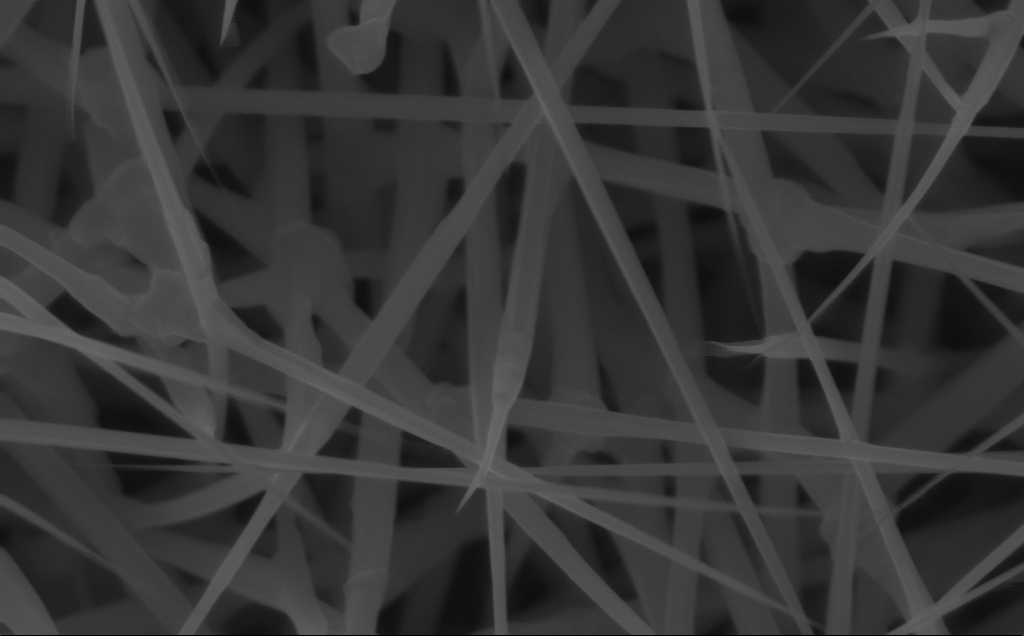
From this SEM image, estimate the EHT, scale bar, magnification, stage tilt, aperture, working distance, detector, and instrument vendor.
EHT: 10 kV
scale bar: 200 nm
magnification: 100 K X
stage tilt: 0°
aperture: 30 µm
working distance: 6 mm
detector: InLens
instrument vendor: Zeiss